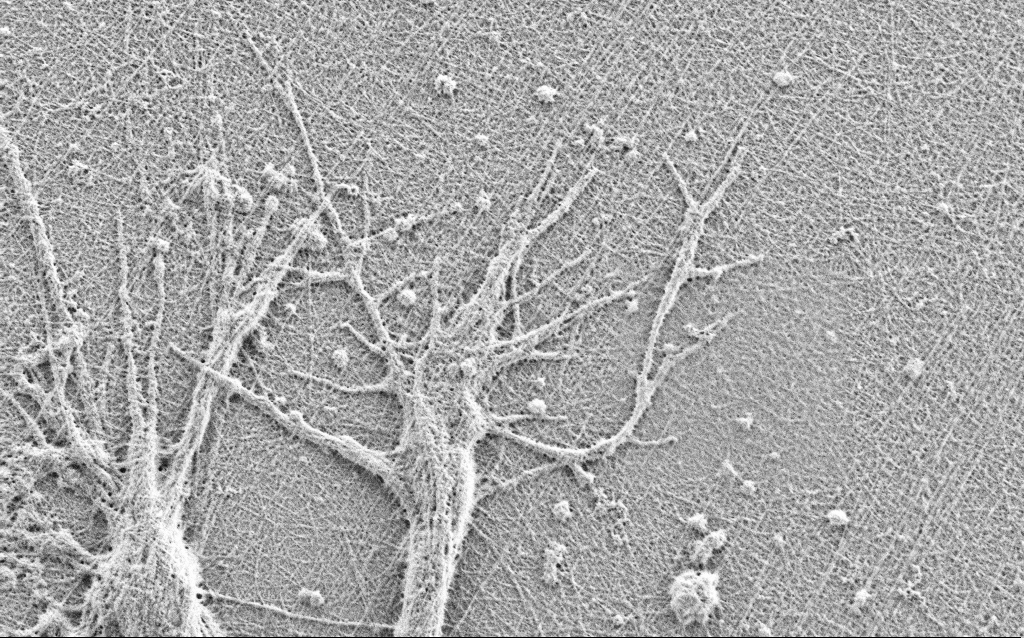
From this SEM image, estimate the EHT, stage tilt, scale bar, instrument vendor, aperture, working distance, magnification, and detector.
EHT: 0.9 kV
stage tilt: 0°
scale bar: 2000 nm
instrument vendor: Zeiss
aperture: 30 µm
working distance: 3 mm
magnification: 15 K X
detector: SE2